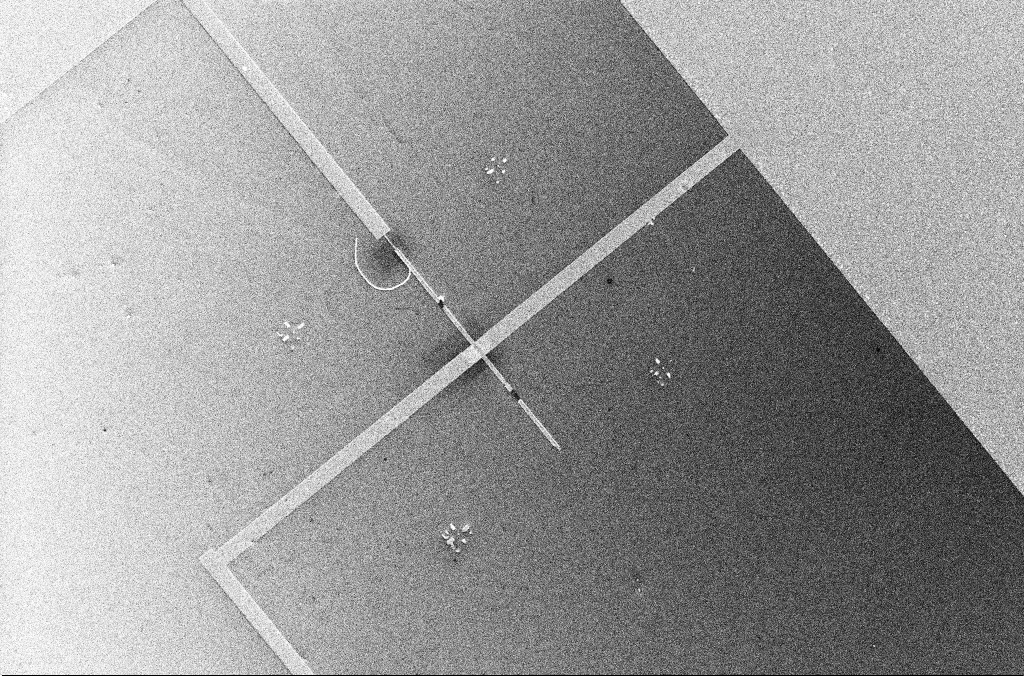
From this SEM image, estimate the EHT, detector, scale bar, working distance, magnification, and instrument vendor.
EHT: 5 kV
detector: InLens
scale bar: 20000 nm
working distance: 3.4 mm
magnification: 1.22 K X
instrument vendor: Zeiss